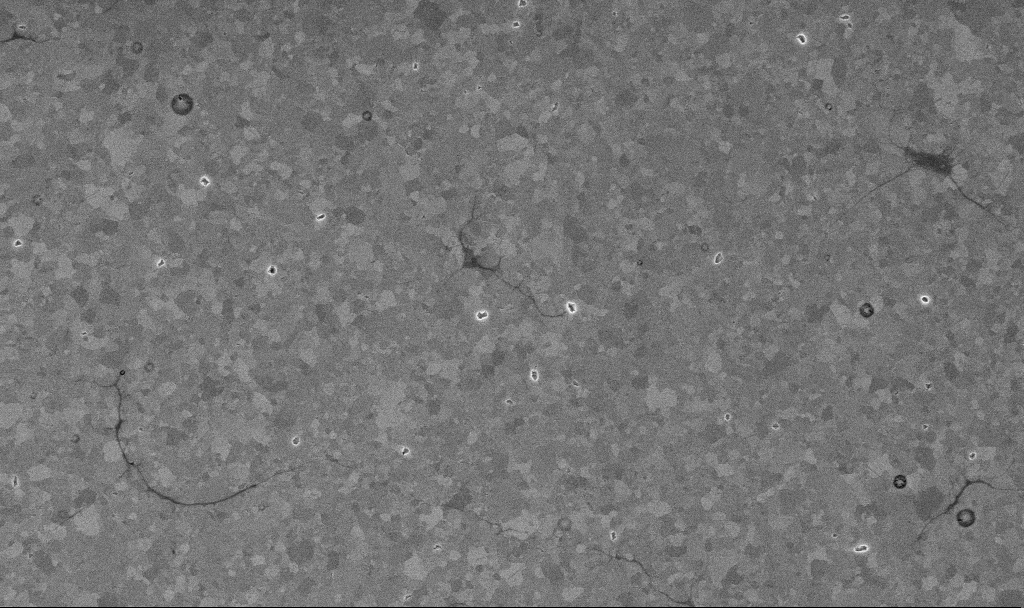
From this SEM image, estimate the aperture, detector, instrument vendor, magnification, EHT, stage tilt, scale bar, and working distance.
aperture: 30 µm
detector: InLens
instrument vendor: Zeiss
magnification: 20.87 K X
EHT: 10 kV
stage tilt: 0°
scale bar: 1000 nm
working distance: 3.3 mm